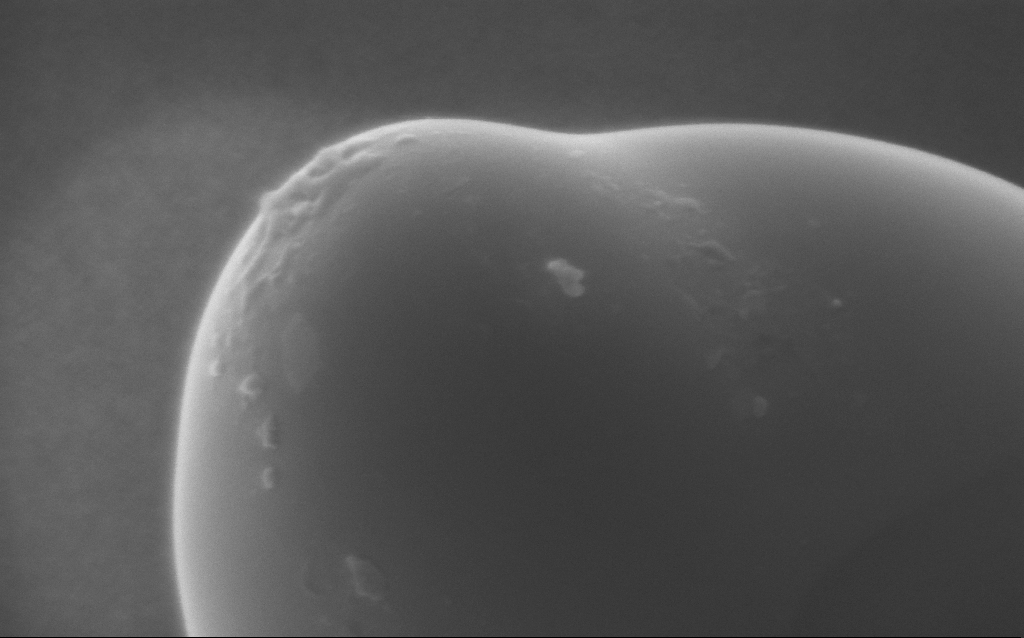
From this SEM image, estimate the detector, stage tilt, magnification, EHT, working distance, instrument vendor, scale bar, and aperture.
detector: InLens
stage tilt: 0°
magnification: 269.09 K X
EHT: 5 kV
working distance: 3 mm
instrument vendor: Zeiss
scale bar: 200 nm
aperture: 30 µm